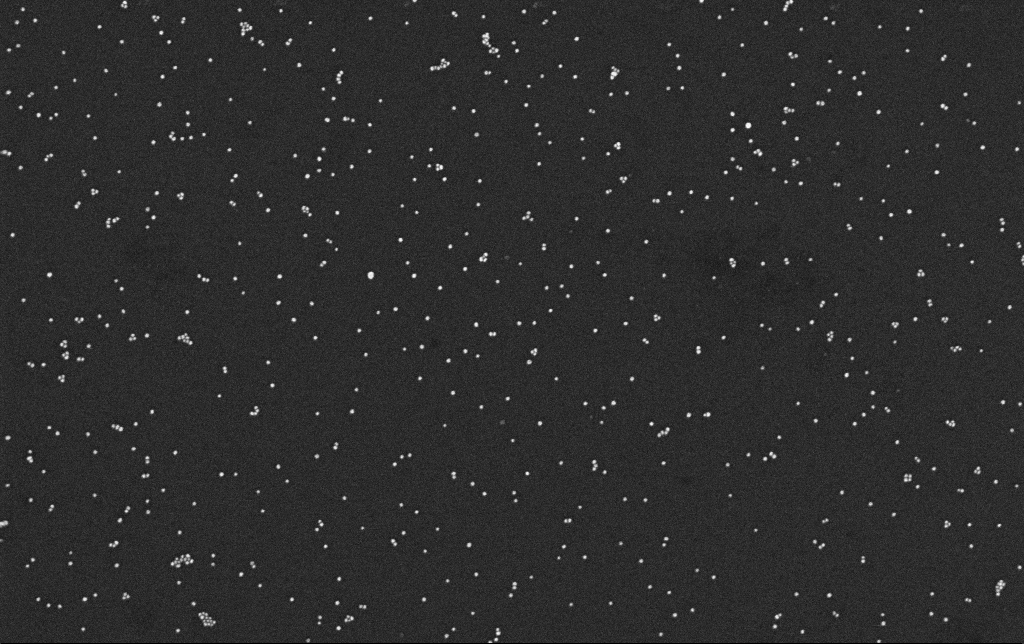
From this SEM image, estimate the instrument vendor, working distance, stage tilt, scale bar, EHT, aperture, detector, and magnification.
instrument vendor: Zeiss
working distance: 3.4 mm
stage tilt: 0°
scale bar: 200 nm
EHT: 10 kV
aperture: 30 µm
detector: InLens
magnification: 100 K X